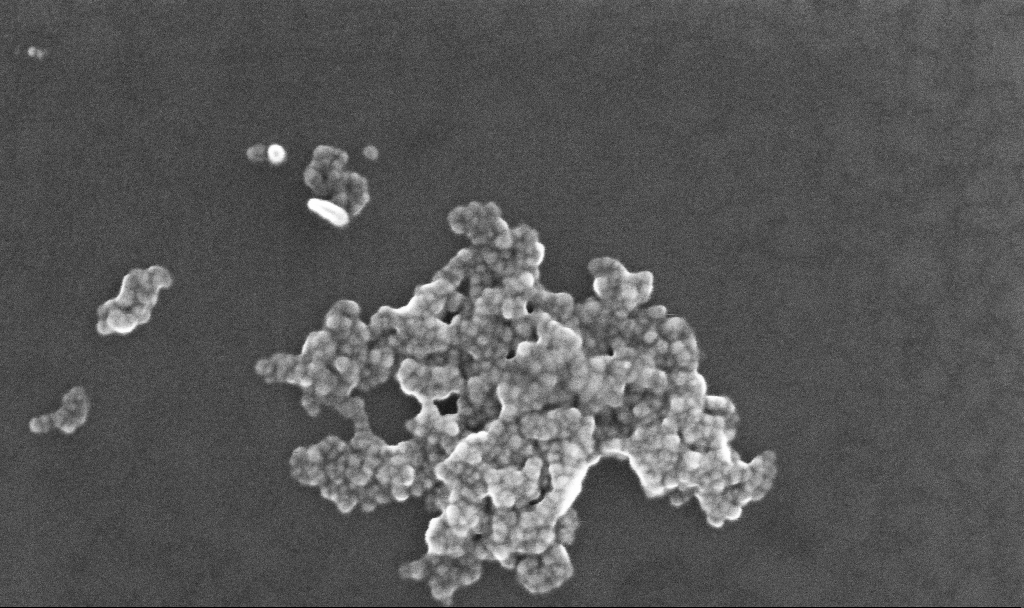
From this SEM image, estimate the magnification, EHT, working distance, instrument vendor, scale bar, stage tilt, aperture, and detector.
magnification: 182.1 K X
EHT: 10 kV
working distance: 5.2 mm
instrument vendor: Zeiss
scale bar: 200 nm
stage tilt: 0°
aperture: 30 µm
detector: InLens